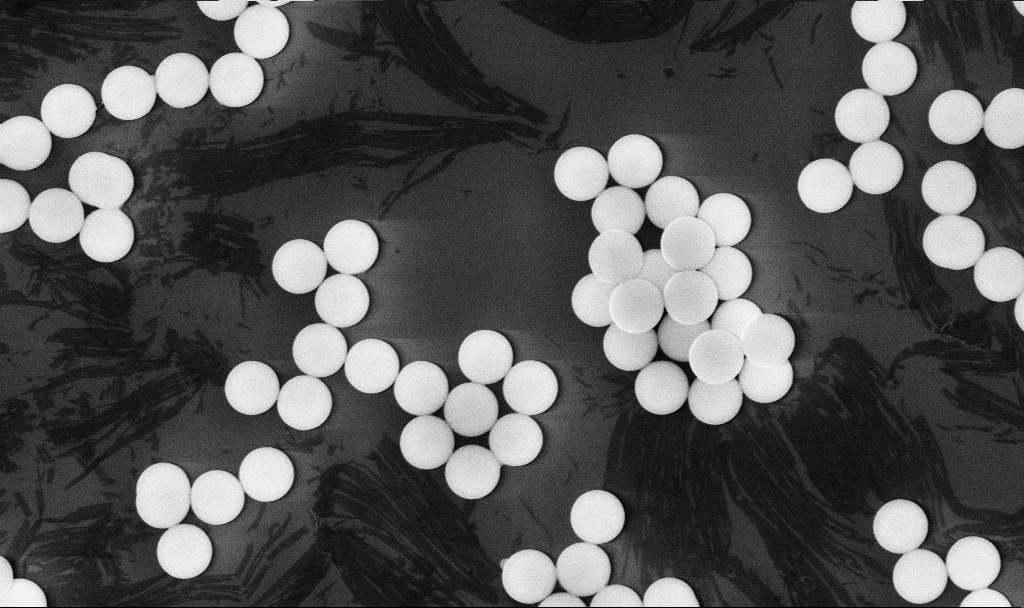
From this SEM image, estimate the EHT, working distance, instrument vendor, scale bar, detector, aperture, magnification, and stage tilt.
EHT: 10 kV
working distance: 5.4 mm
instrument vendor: Zeiss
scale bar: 2000 nm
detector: InLens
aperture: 30 µm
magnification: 15.6 K X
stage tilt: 0°